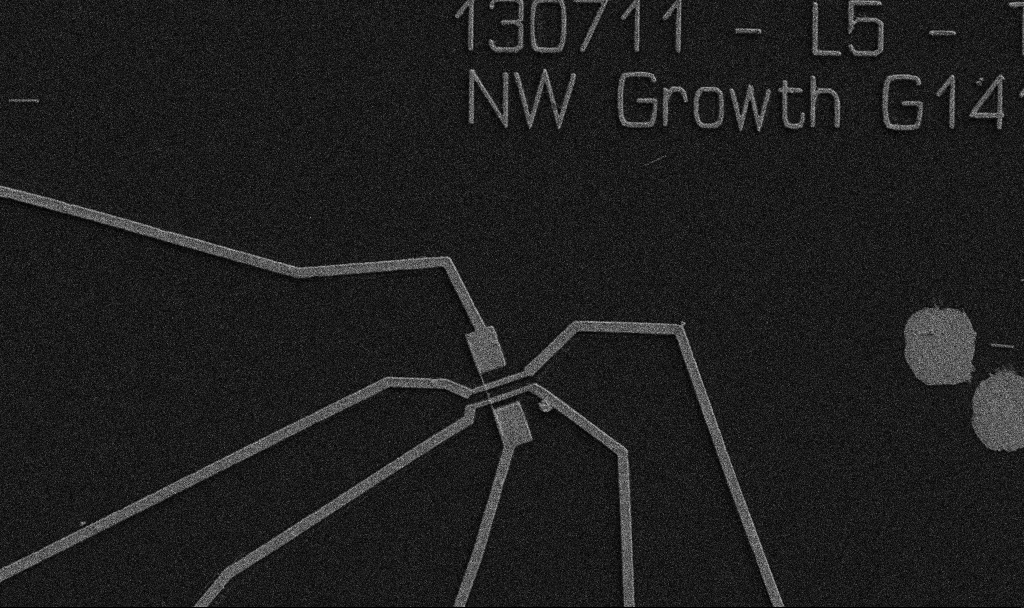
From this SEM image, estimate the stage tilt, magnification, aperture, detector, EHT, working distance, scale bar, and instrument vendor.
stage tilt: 0°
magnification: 5 K X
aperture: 30 µm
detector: SE2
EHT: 5 kV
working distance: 10.7 mm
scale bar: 10000 nm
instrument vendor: Zeiss